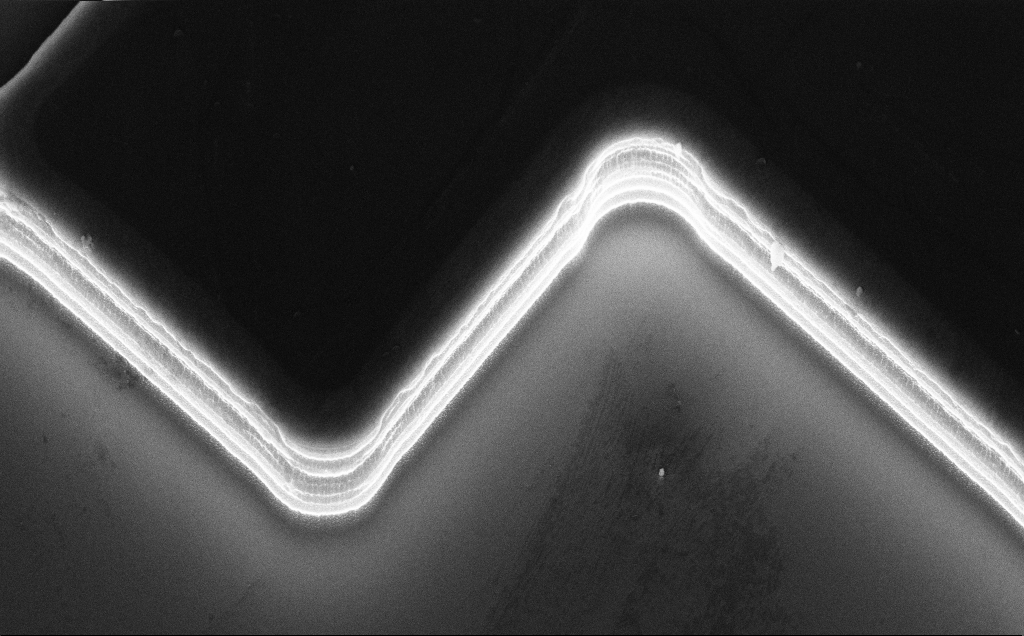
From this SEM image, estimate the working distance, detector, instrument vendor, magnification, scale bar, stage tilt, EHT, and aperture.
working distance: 9 mm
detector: InLens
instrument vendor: Zeiss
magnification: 9.99 K X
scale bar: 2000 nm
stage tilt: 50°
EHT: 10 kV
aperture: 30 µm